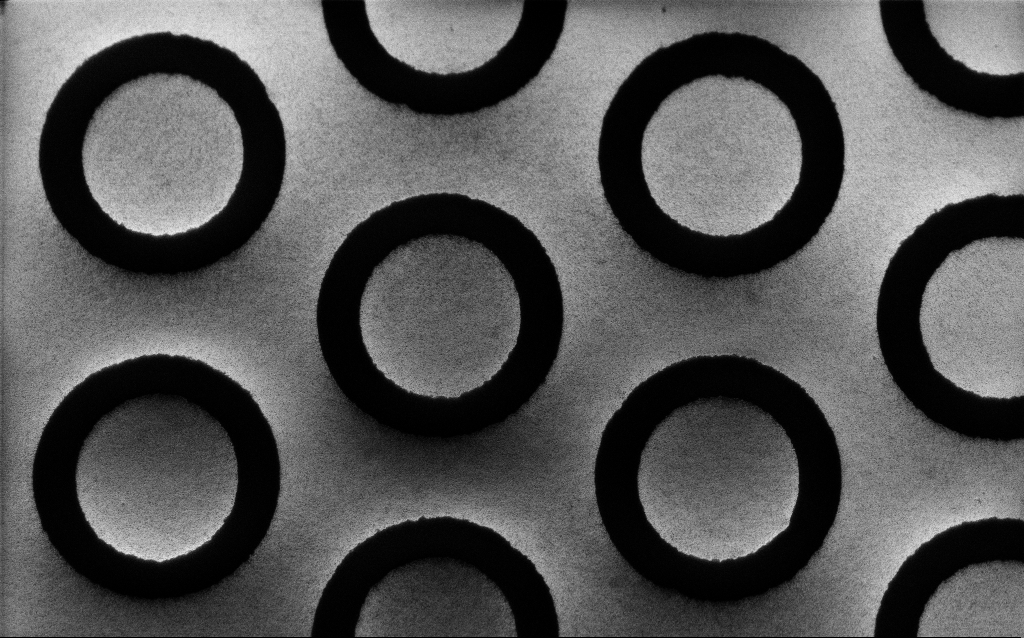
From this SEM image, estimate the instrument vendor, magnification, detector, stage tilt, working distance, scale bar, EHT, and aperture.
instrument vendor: Zeiss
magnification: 0.742 K X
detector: InLens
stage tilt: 45°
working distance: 7 mm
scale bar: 20000 nm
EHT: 20 kV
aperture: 30 µm